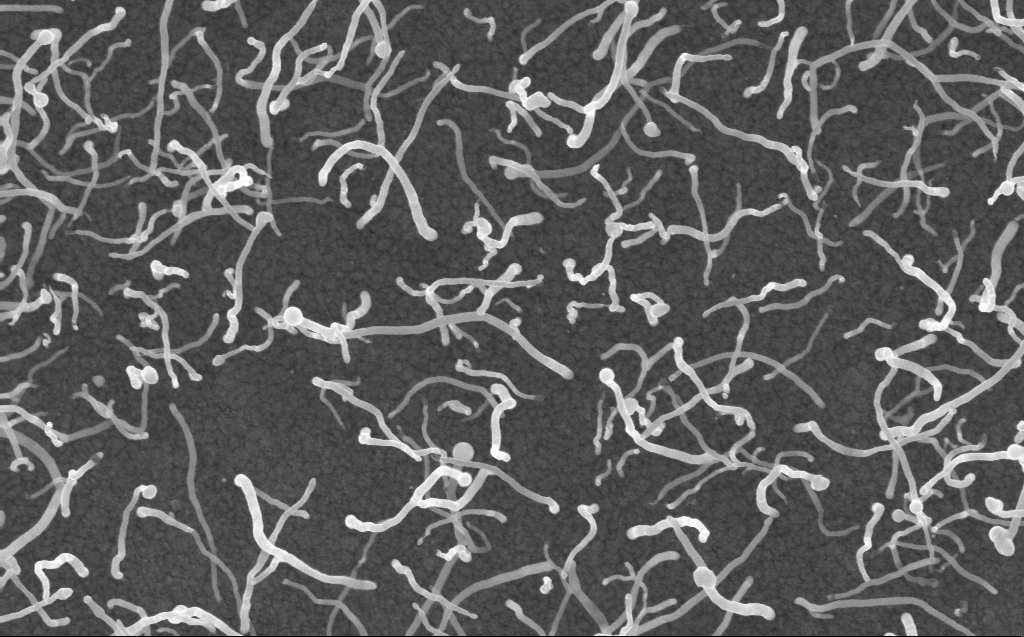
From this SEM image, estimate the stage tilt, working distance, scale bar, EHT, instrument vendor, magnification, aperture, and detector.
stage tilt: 0°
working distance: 3 mm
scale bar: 1000 nm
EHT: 10 kV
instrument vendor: Zeiss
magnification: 50 K X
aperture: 30 µm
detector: InLens